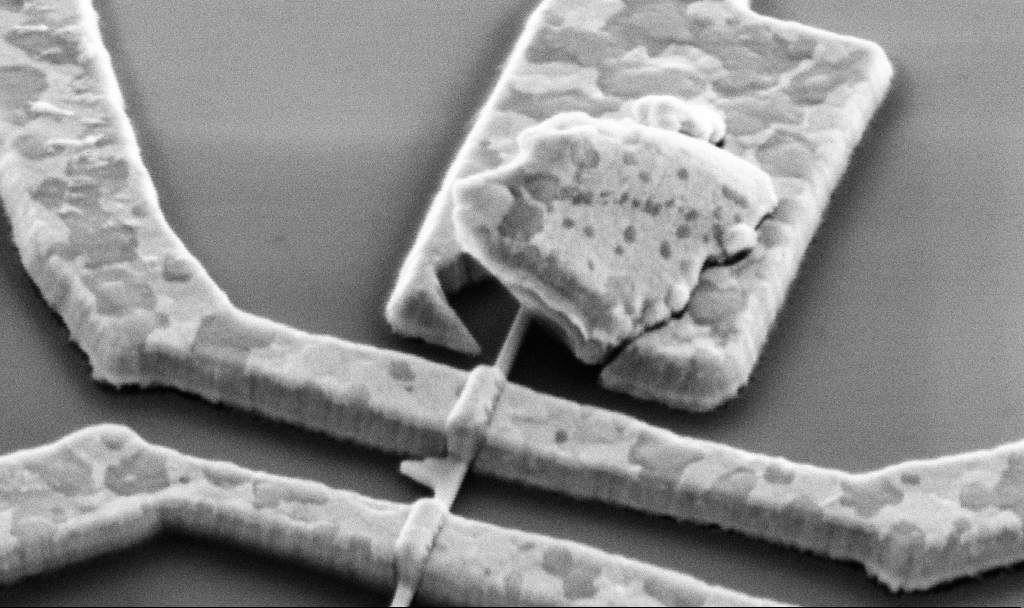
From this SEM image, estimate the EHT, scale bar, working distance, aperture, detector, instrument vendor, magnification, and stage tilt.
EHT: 5 kV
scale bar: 1000 nm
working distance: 14.7 mm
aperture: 30 µm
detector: SE2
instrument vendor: Zeiss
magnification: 60 K X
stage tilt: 45°